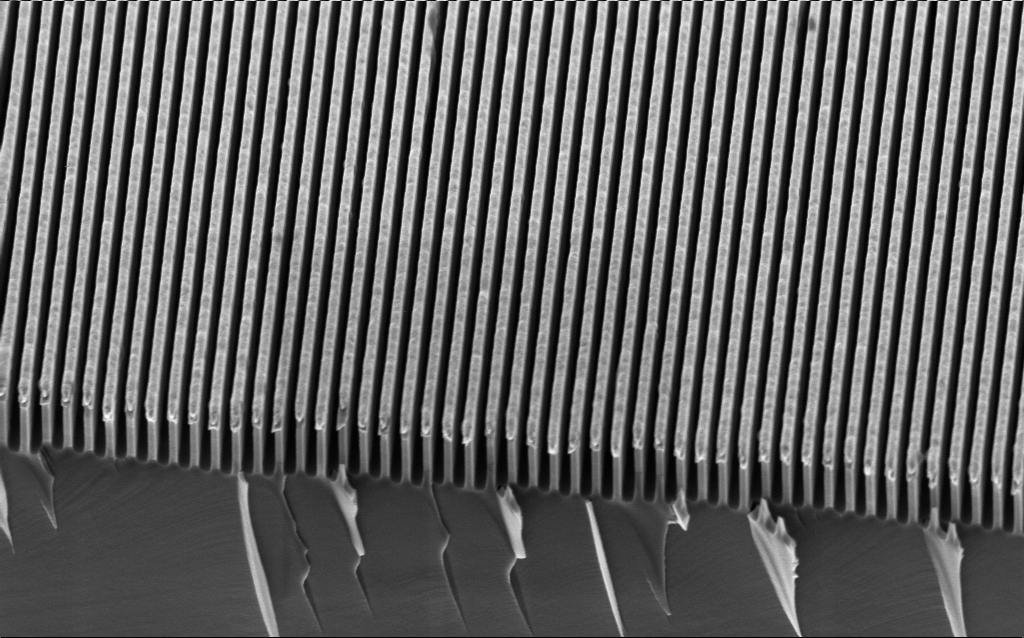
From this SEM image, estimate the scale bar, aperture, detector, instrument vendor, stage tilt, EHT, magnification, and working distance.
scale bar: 1000 nm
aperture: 30 µm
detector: InLens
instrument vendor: Zeiss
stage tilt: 45°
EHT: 2 kV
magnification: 43.09 K X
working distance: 3.2 mm